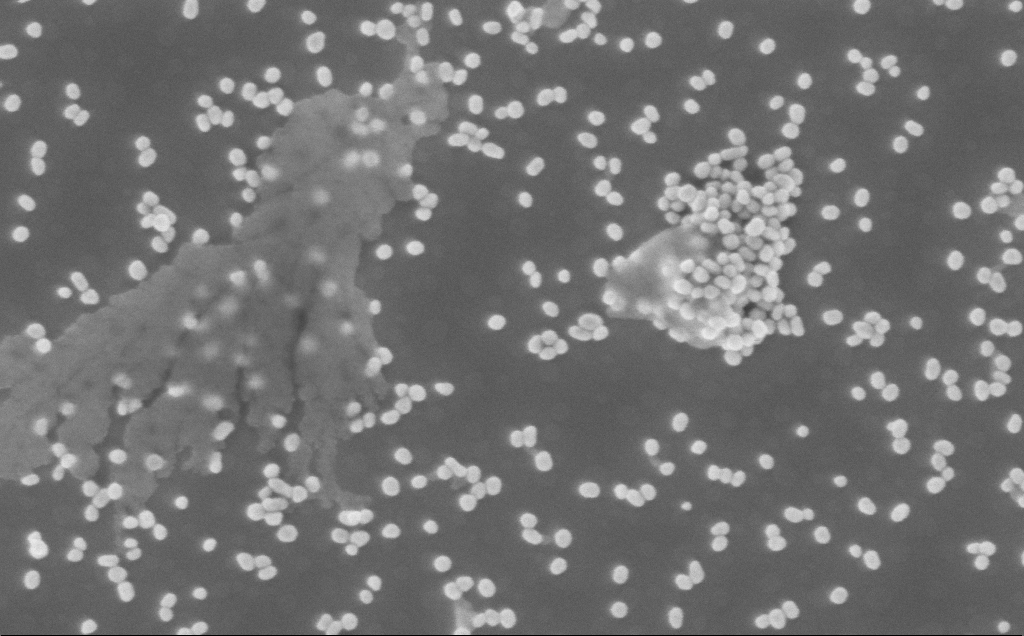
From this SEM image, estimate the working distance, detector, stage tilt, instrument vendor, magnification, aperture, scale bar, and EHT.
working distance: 5 mm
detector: InLens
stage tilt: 0°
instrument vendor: Zeiss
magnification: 150 K X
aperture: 30 µm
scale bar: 100 nm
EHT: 3 kV